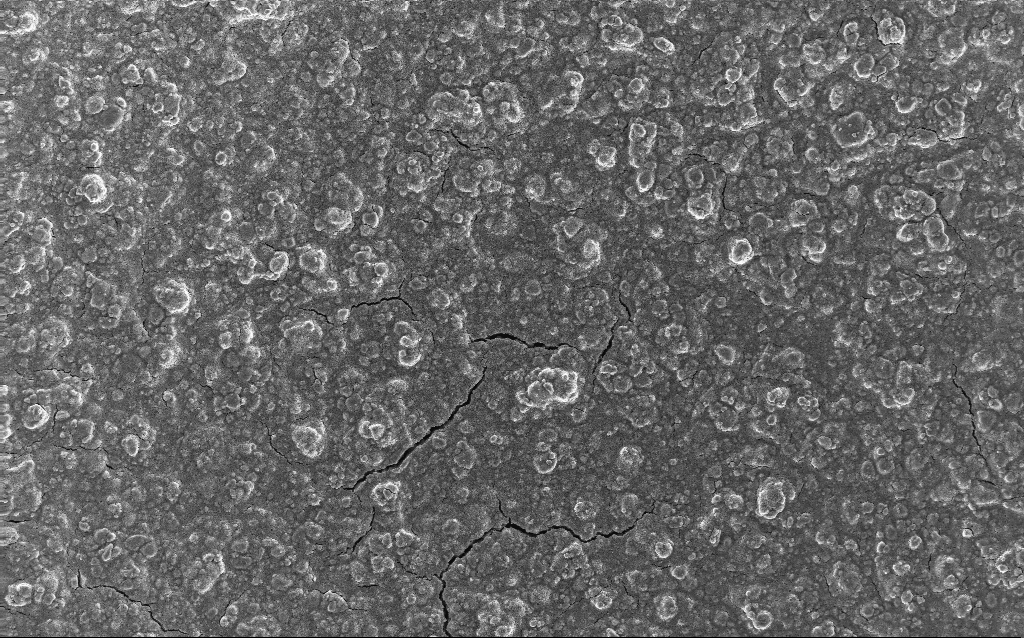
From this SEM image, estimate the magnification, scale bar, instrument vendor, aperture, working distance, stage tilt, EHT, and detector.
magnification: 0.77 K X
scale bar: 20000 nm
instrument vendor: Zeiss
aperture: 30 µm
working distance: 2.6 mm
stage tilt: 0°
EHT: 10 kV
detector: InLens